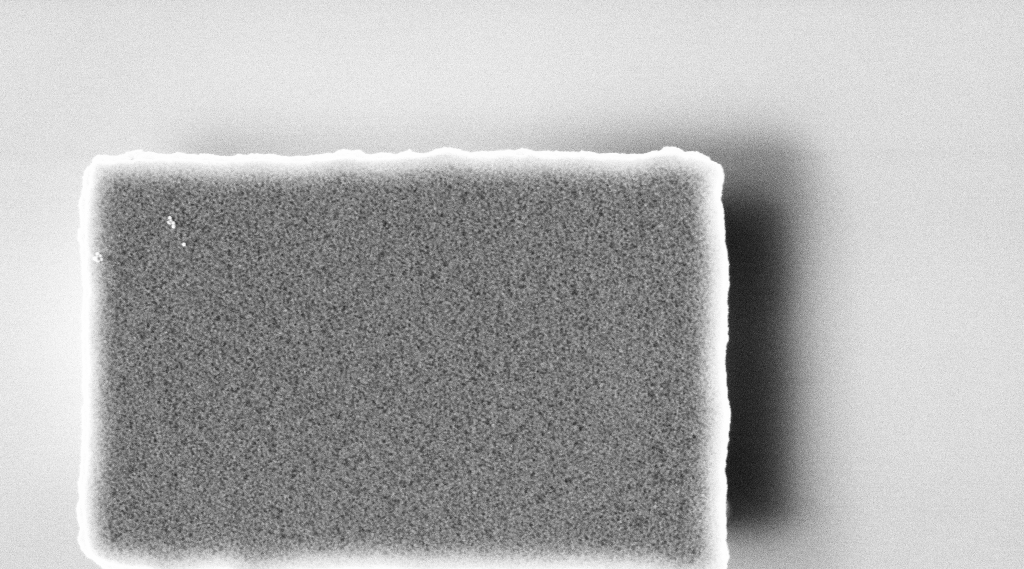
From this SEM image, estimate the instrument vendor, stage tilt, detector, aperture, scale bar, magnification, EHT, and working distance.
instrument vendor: Zeiss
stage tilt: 0°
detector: InLens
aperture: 30 µm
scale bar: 200 nm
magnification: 80.16 K X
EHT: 3 kV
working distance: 2.5 mm